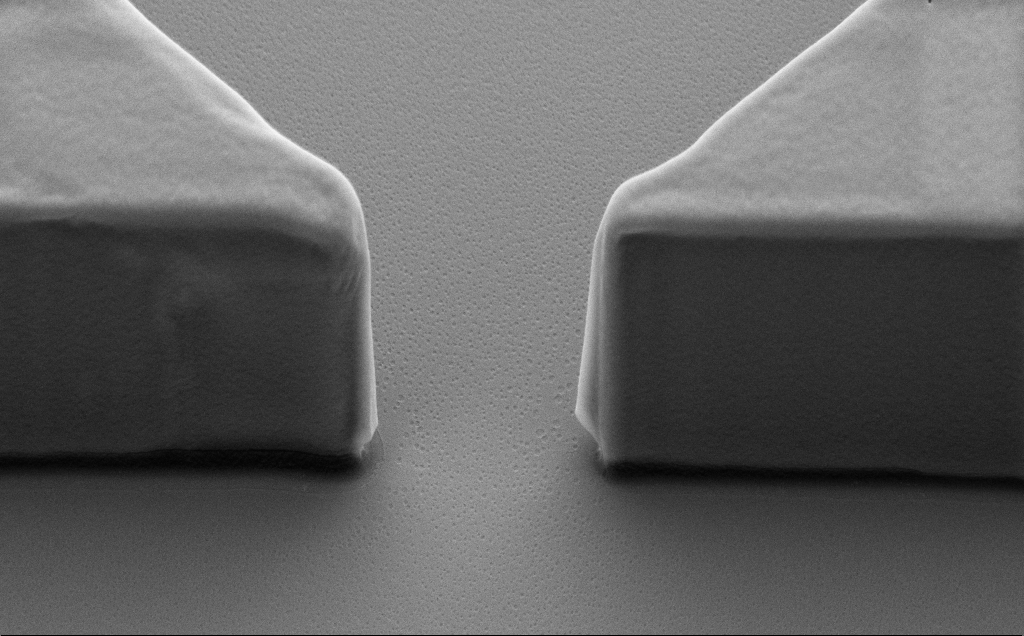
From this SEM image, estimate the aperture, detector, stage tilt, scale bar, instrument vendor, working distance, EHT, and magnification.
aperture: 30 µm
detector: SE2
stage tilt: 40°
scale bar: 1000 nm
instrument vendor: Zeiss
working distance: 9 mm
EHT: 5 kV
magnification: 15 K X